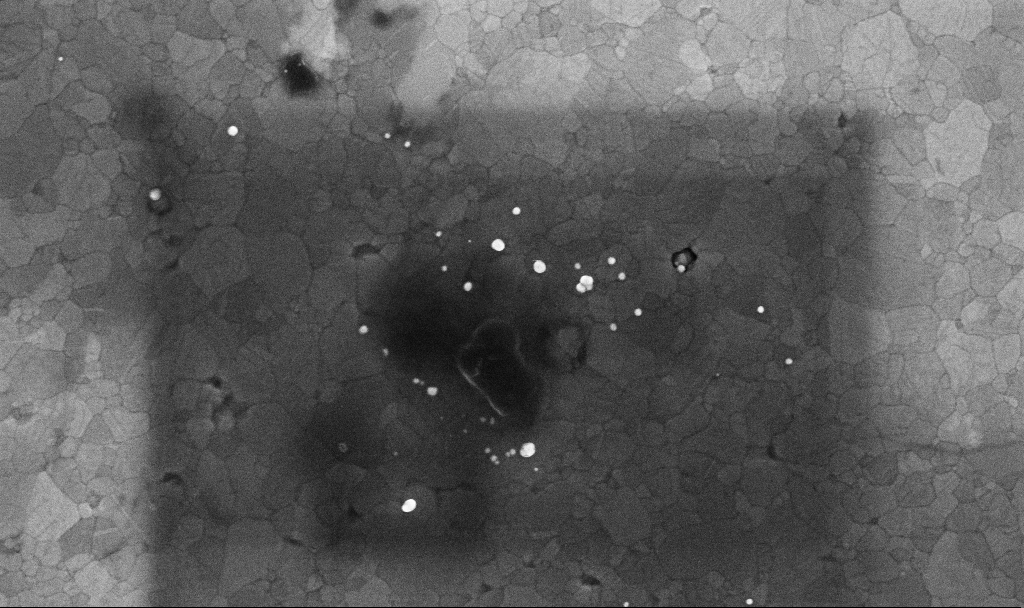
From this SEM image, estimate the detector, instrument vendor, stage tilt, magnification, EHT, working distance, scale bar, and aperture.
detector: InLens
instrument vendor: Zeiss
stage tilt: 0°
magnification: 70.32 K X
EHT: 10 kV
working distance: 3.4 mm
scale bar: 1000 nm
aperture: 30 µm